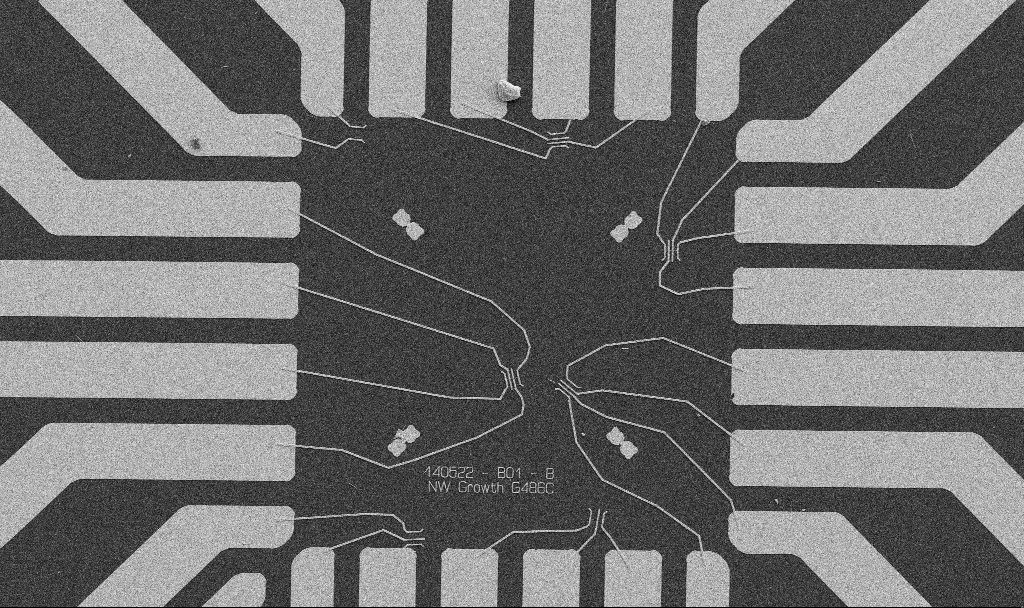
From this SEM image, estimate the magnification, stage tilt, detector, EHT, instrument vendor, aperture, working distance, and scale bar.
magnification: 1 K X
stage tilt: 0°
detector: SE2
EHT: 5 kV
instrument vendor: Zeiss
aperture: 30 µm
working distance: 10.6 mm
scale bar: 20000 nm